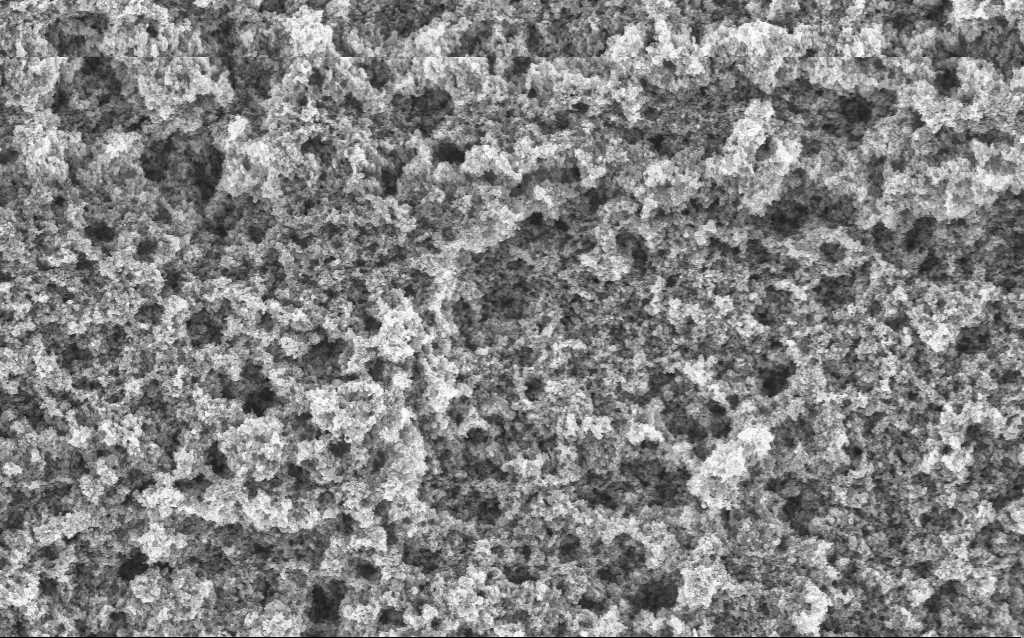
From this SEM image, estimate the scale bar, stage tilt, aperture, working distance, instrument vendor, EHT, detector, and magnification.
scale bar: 1000 nm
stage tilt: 0°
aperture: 30 µm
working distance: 4.4 mm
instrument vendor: Zeiss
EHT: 5 kV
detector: InLens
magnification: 37.88 K X